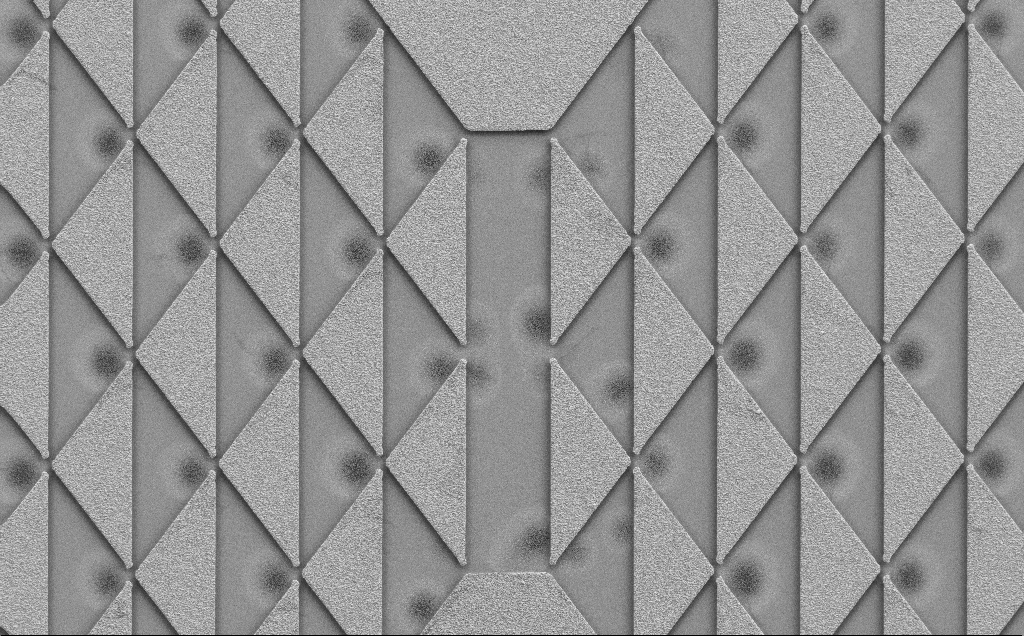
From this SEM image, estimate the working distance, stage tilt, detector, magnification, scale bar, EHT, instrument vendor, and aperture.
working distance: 6 mm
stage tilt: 0°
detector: SE2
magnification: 0.871 K X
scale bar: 20000 nm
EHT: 5 kV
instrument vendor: Zeiss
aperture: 30 µm